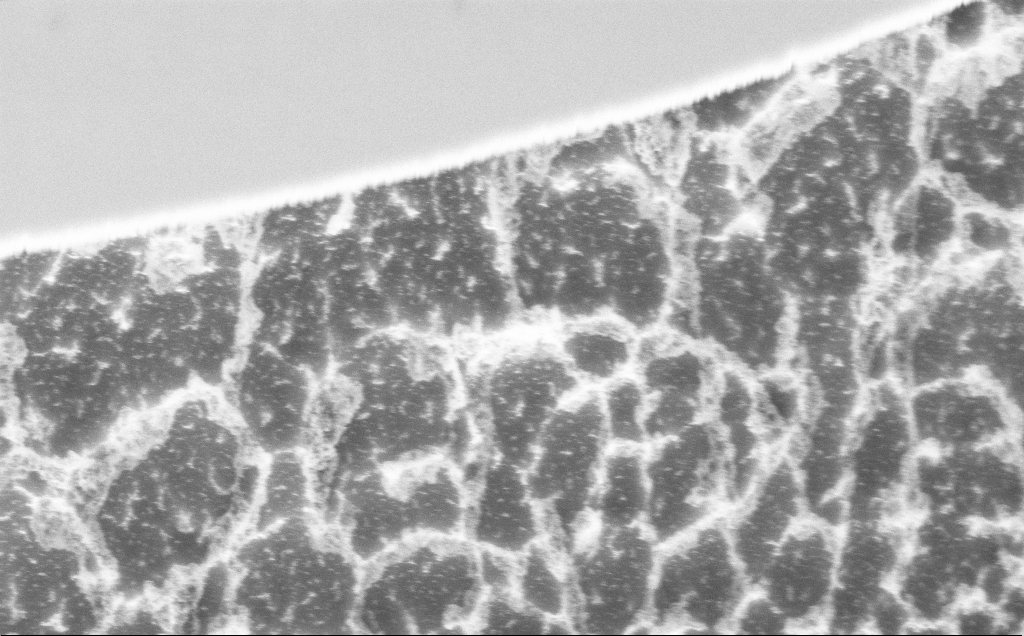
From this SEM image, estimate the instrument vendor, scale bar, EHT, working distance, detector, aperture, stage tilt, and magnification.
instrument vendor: Zeiss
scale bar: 2000 nm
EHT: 5 kV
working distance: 8 mm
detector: InLens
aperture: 30 µm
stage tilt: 45°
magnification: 33.68 K X